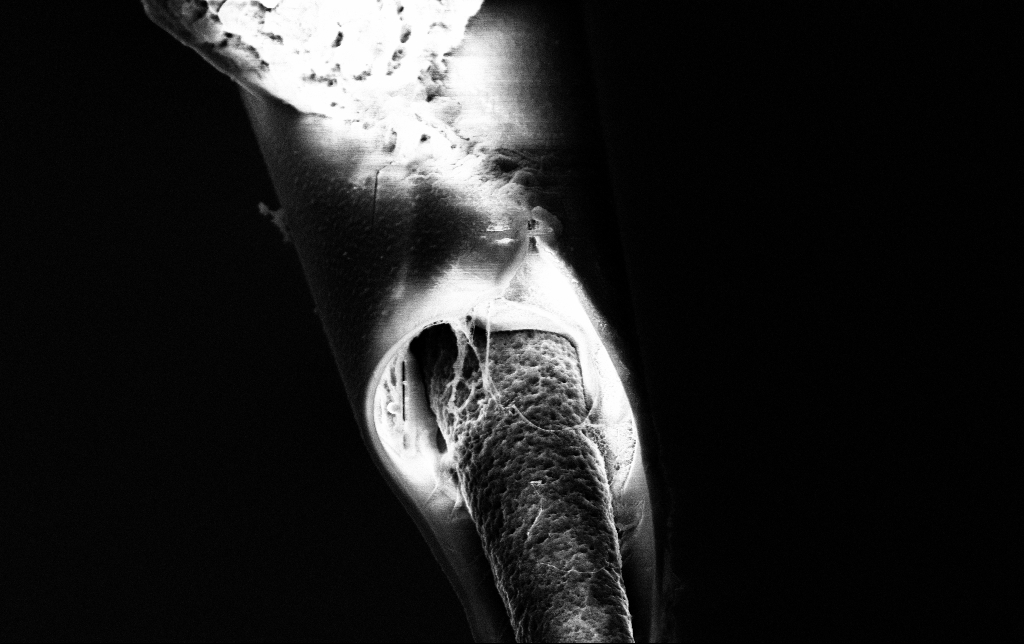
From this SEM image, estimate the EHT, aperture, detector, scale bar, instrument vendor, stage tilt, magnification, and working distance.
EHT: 3 kV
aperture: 30 µm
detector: InLens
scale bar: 2000 nm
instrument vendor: Zeiss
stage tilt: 45°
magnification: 25 K X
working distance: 7.3 mm